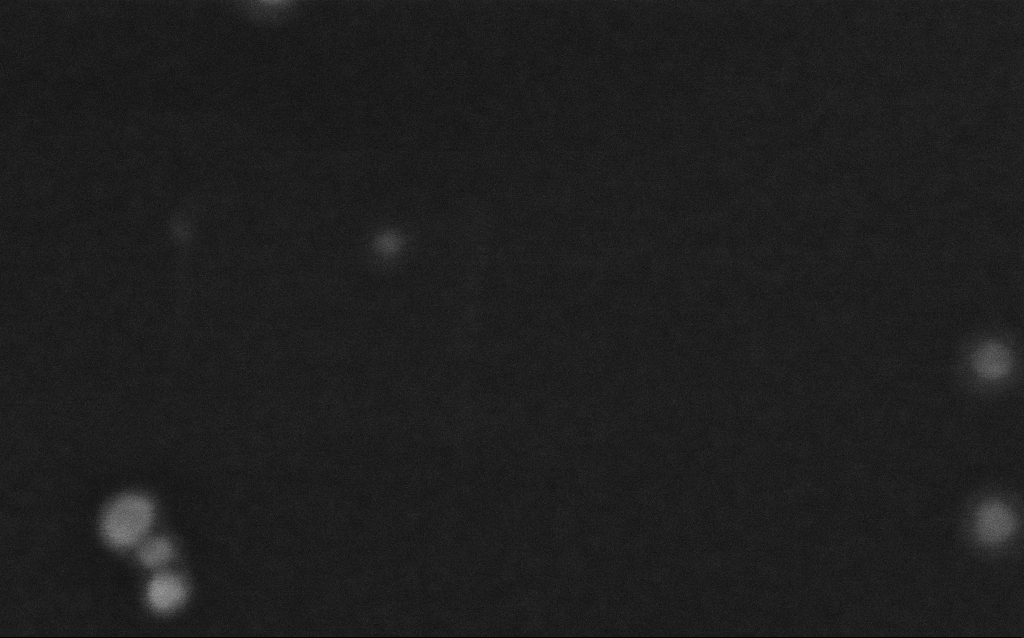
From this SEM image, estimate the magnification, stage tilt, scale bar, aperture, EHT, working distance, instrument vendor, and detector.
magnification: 1137.06 K X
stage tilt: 0°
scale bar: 20 nm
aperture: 30 µm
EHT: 8 kV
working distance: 2.7 mm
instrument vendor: Zeiss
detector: InLens